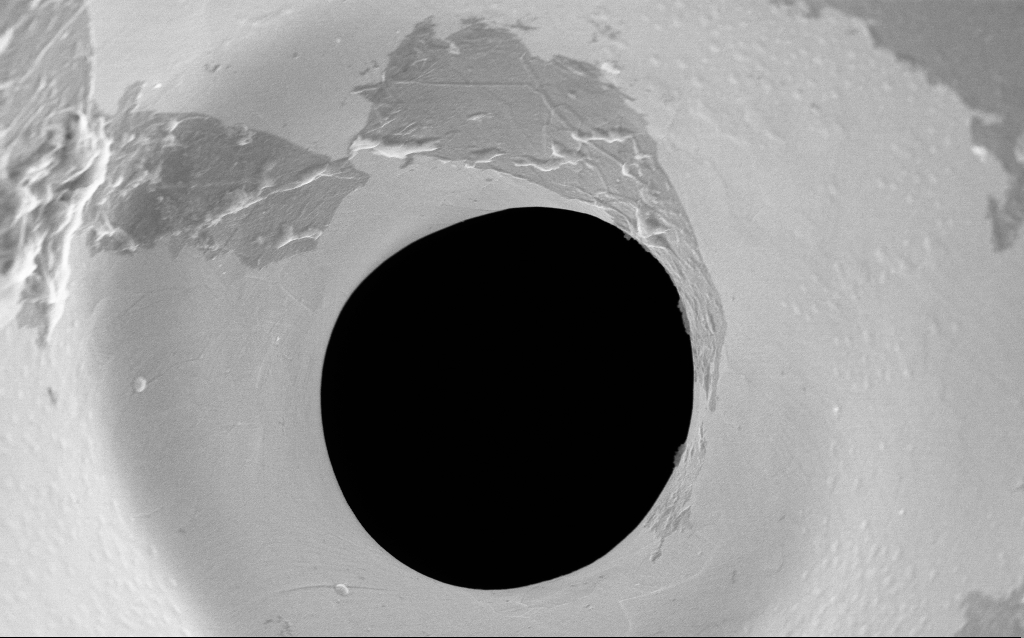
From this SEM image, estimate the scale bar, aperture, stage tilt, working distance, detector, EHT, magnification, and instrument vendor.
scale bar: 2000 nm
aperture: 30 µm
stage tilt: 0°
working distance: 4.3 mm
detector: InLens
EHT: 5 kV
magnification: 31.36 K X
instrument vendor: Zeiss